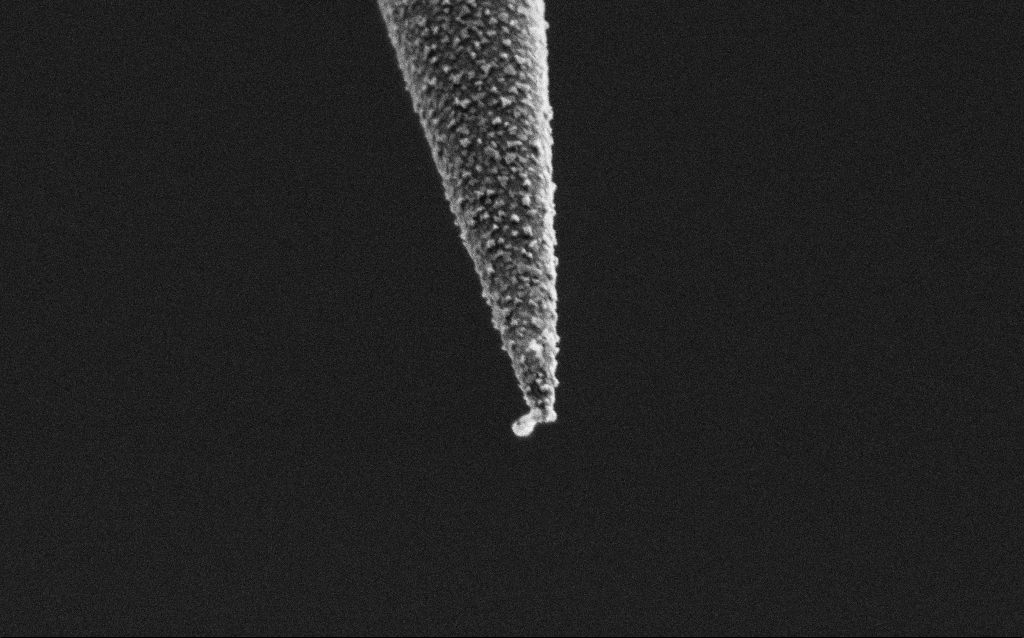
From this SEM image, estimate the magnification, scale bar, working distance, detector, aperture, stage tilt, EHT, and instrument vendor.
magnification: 50 K X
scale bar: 1000 nm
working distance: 7.6 mm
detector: SE2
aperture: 30 µm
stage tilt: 45°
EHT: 2 kV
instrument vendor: Zeiss